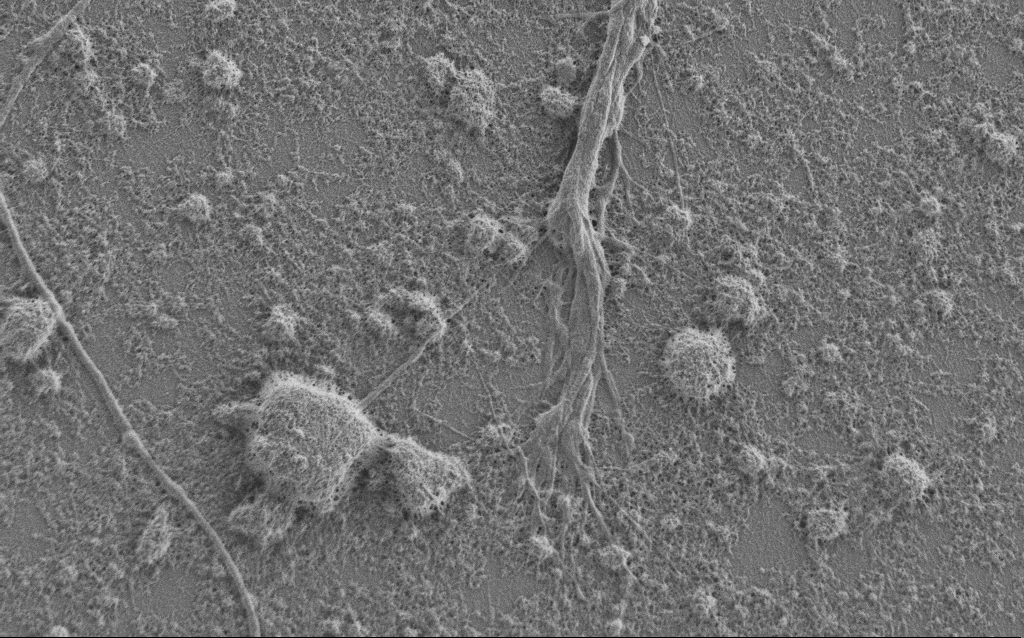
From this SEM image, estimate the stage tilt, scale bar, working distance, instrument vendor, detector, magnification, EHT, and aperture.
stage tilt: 0°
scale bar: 2000 nm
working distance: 6 mm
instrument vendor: Zeiss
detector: SE2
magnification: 7.5 K X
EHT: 1 kV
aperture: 30 µm